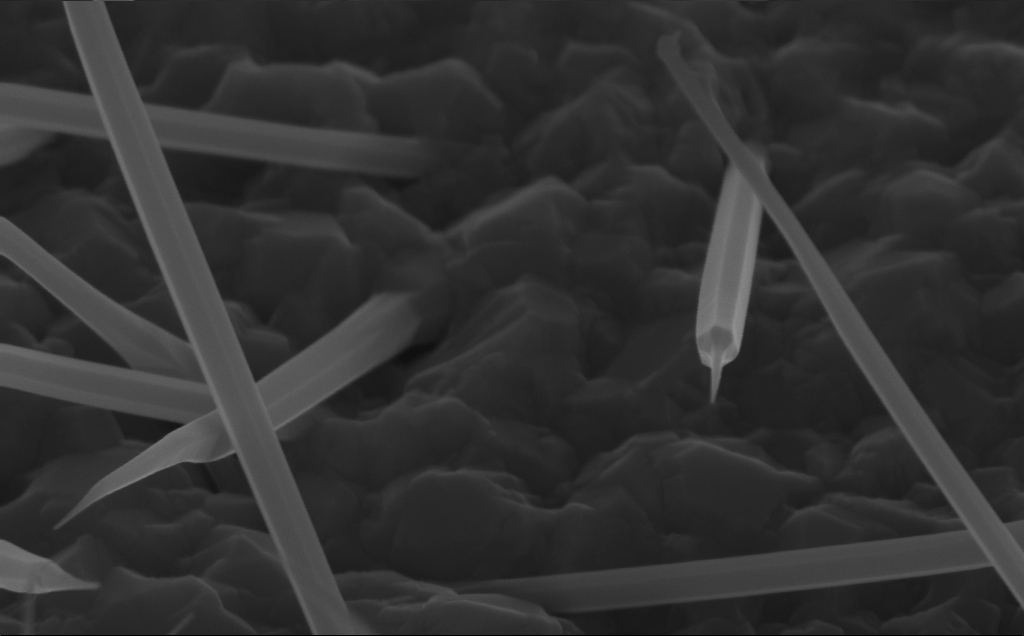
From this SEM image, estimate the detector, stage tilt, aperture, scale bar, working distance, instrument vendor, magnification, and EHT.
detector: InLens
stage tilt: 45°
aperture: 30 µm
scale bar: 200 nm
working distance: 5 mm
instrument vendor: Zeiss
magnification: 124.23 K X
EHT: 10 kV